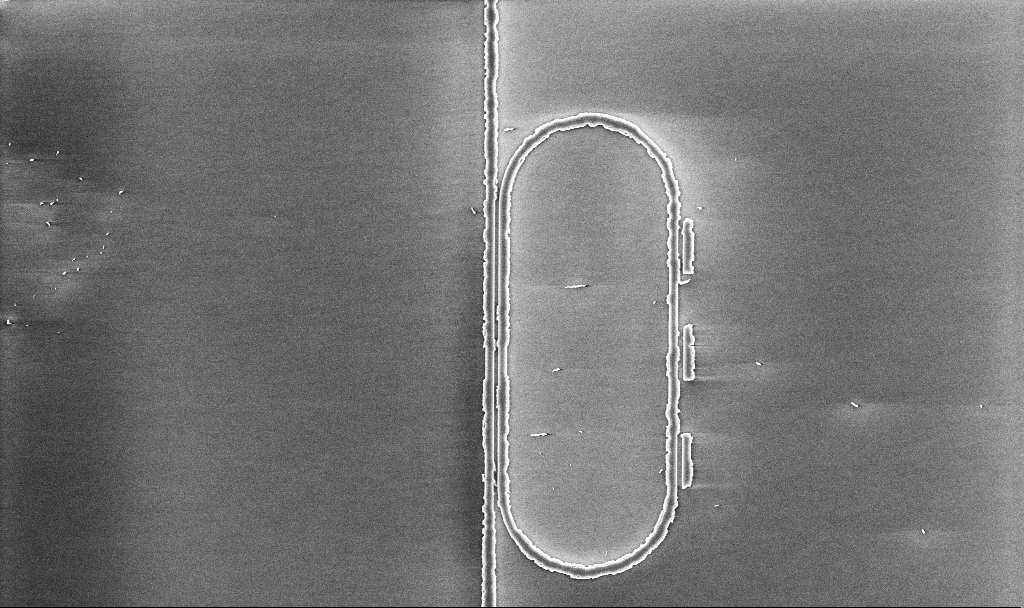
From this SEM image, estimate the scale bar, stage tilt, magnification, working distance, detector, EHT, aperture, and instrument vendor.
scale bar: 10000 nm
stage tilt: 0°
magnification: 6.63 K X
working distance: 10.1 mm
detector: InLens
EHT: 5 kV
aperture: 30 µm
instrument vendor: Zeiss